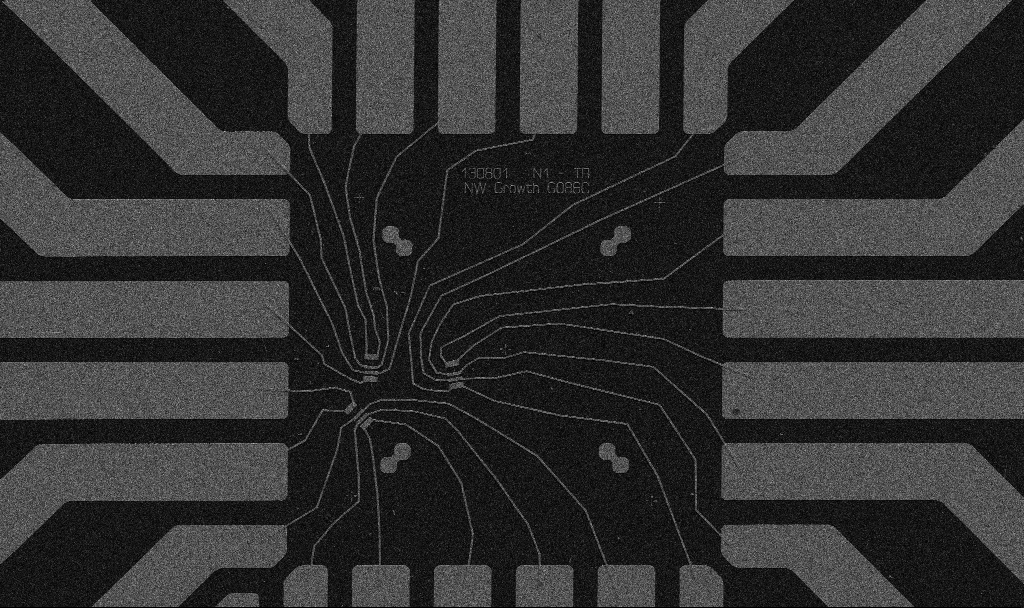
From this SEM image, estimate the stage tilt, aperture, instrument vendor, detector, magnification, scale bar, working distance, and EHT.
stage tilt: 0°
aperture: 30 µm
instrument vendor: Zeiss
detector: SE2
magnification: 1 K X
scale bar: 20000 nm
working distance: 10.7 mm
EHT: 5 kV